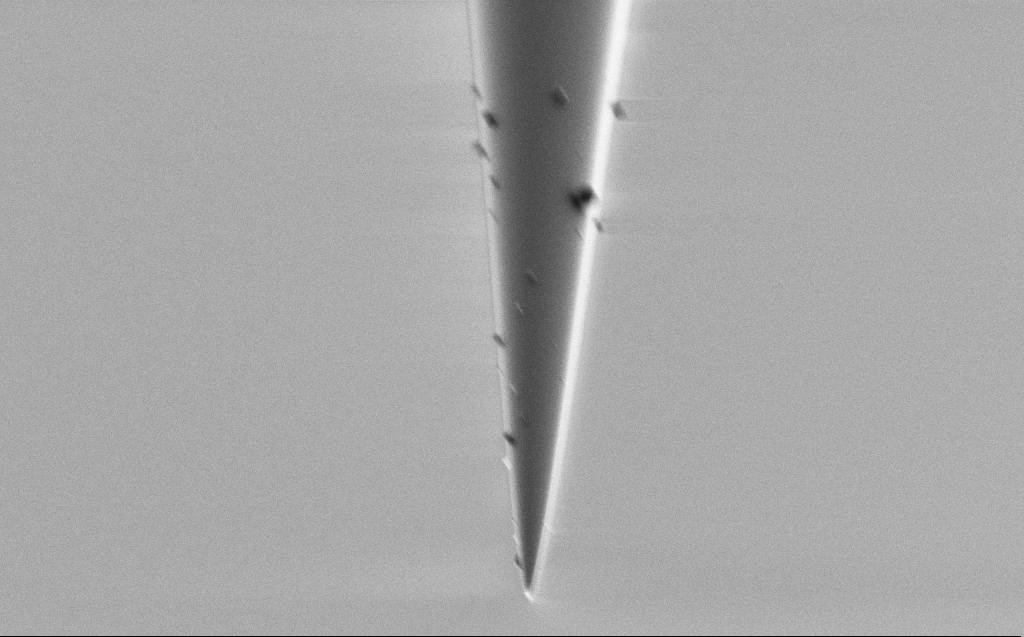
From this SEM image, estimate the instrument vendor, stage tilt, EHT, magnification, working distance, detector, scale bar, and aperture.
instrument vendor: Zeiss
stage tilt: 45.1°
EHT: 1 kV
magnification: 16.52 K X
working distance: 3 mm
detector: SE2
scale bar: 2000 nm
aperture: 30 µm